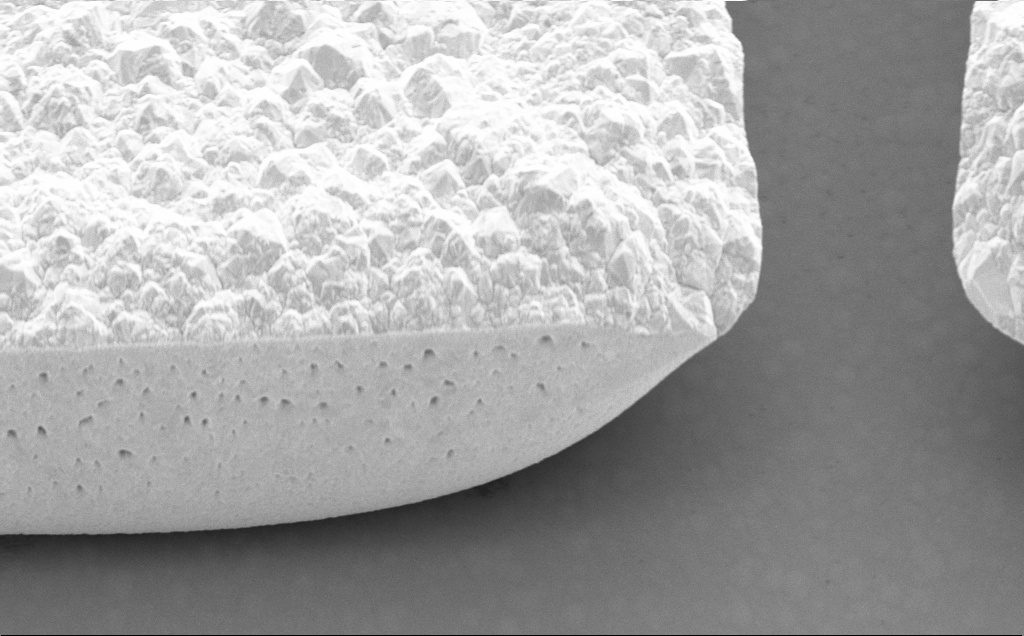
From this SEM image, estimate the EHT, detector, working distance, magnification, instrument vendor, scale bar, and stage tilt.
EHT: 5 kV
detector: SE2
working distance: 14 mm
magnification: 11.31 K X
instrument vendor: Zeiss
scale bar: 2000 nm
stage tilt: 45°